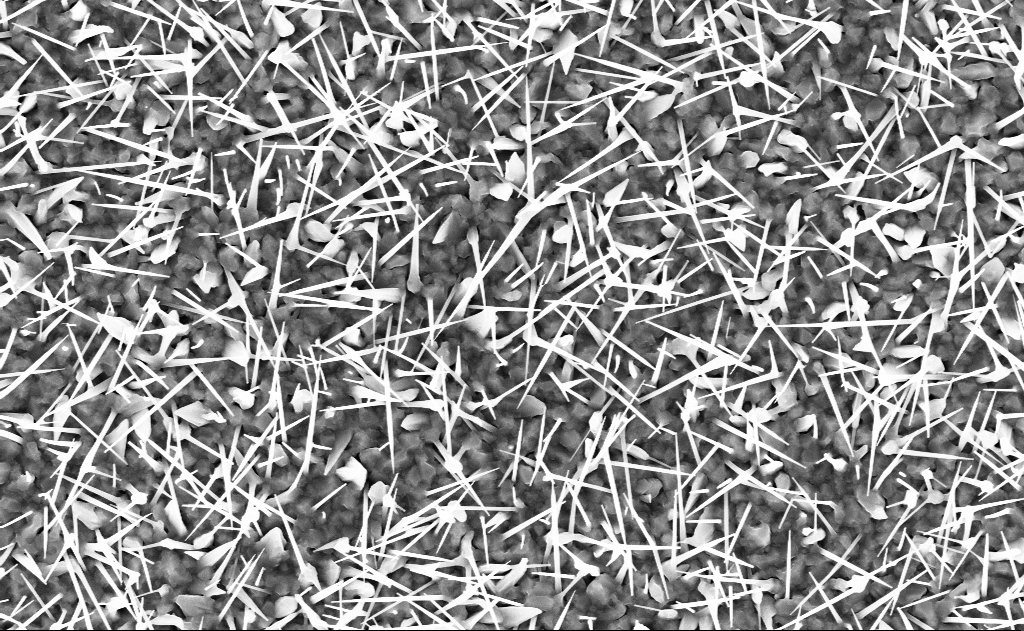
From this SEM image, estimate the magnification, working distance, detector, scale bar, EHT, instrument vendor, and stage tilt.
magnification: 20 K X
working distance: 9 mm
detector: InLens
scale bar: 2000 nm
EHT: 10 kV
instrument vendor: Zeiss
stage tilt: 0°